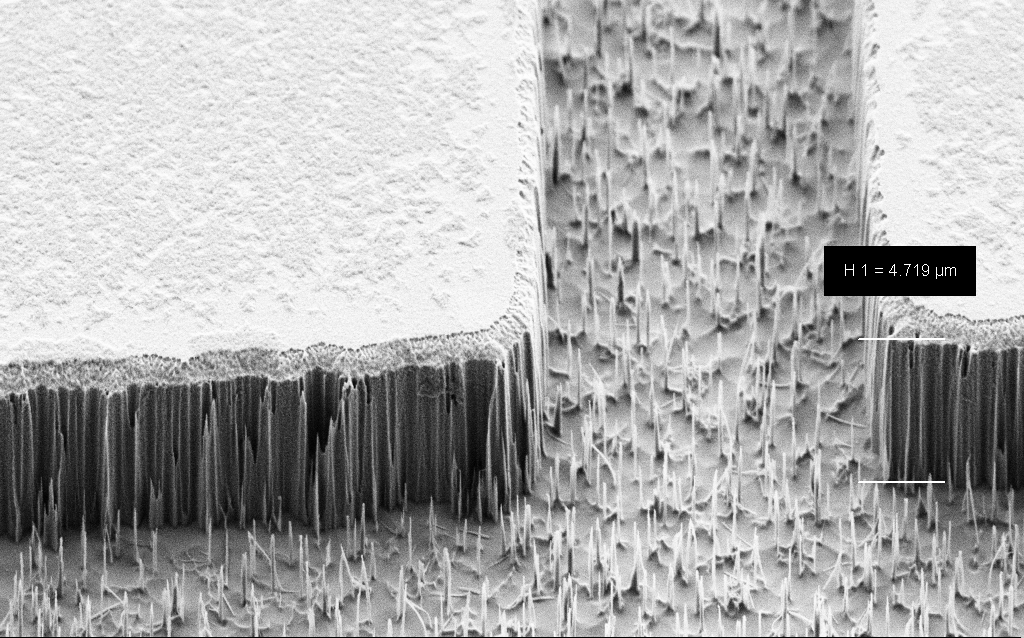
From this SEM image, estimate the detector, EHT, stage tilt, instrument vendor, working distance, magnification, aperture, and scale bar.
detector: SE2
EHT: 2 kV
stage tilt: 45°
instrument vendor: Zeiss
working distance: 8 mm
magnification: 11.13 K X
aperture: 30 µm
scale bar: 2000 nm